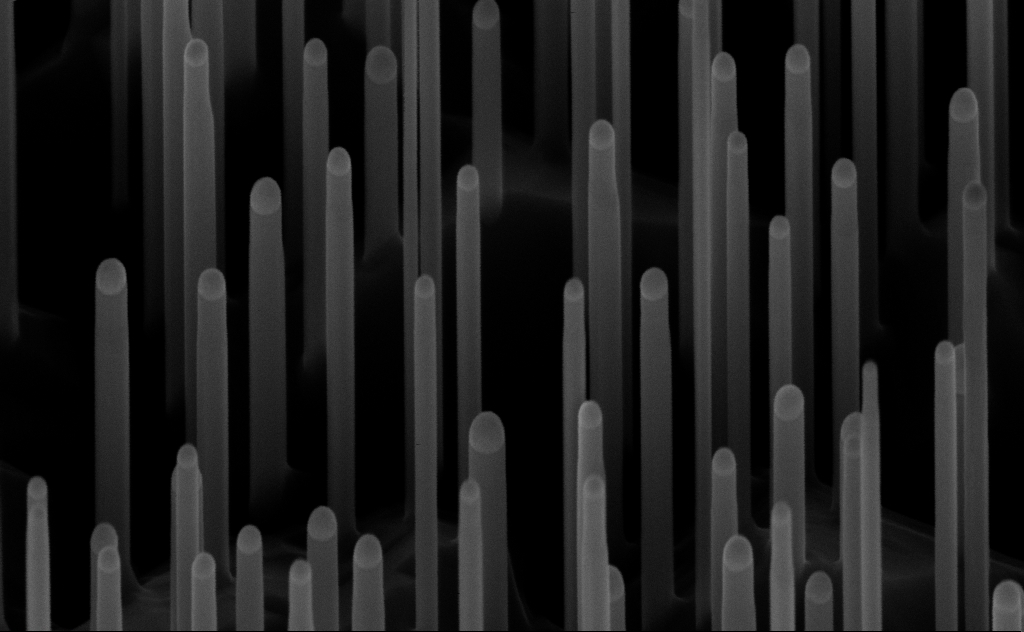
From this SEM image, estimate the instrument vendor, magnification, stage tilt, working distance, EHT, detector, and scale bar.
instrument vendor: Zeiss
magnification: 150 K X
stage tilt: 45°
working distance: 7 mm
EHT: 10 kV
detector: InLens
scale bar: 200 nm